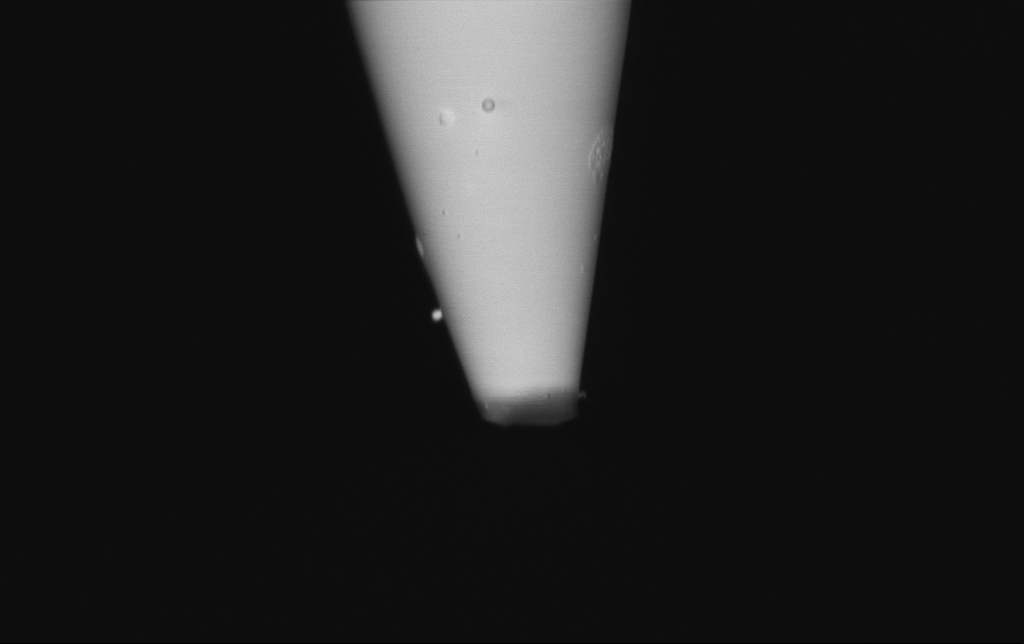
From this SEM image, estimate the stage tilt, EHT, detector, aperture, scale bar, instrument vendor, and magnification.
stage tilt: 0°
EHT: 1 kV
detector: InLens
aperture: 30 µm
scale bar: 2000 nm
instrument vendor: Zeiss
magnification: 25 K X